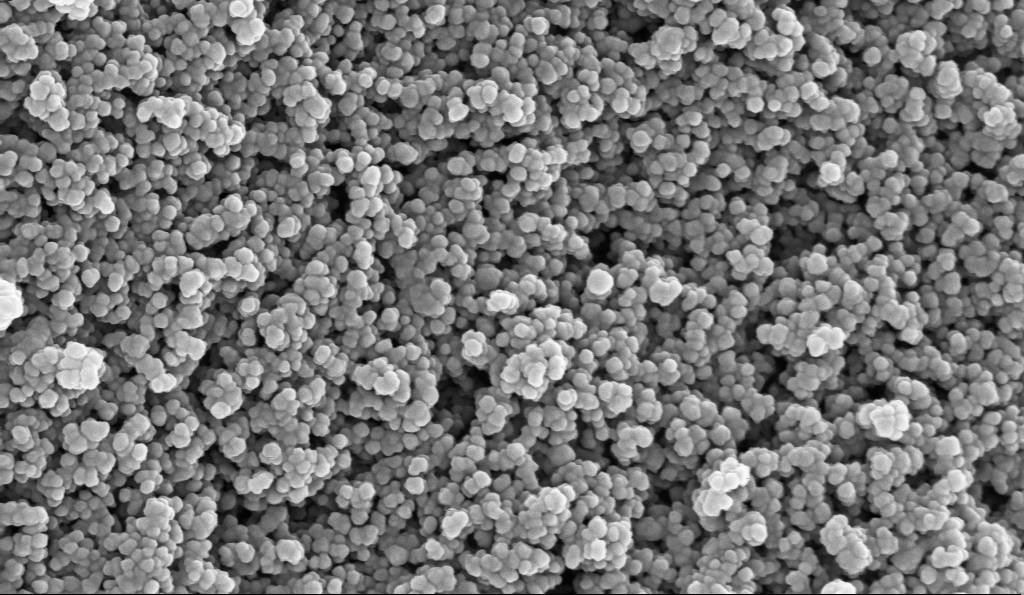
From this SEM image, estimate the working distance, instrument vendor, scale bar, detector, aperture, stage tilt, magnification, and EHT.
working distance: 5.1 mm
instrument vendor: Zeiss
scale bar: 100 nm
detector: InLens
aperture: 30 µm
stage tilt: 0°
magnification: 135 K X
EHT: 10 kV